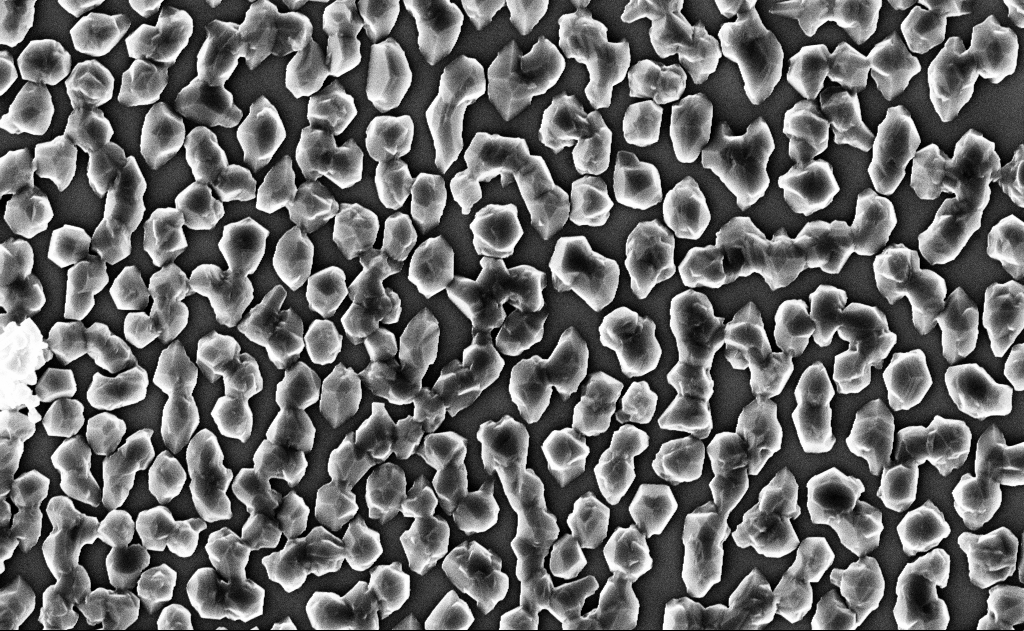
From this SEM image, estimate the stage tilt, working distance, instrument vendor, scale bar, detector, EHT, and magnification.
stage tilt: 0°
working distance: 12 mm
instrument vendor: Zeiss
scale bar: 1000 nm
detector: InLens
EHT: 10 kV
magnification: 20 K X